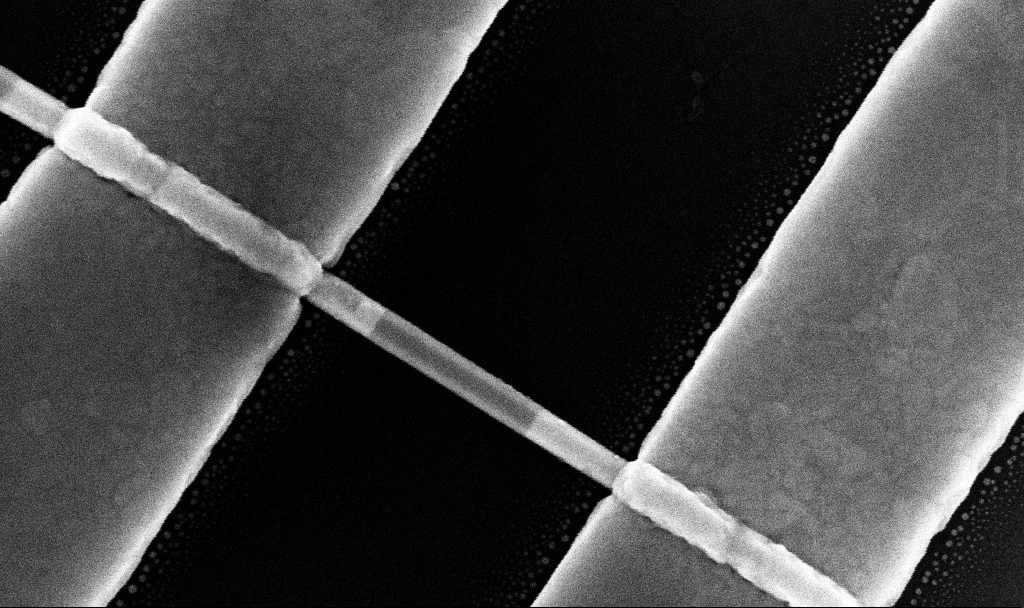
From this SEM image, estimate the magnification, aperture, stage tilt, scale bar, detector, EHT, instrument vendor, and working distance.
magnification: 174.01 K X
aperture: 30 µm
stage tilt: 0°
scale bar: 200 nm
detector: InLens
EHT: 10 kV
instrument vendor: Zeiss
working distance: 7.8 mm